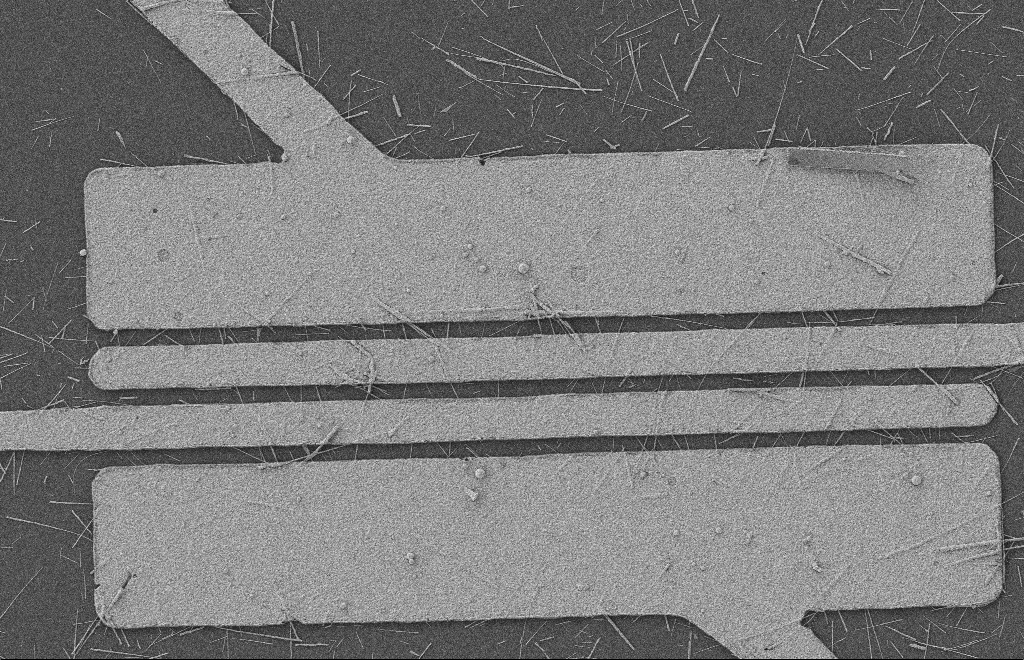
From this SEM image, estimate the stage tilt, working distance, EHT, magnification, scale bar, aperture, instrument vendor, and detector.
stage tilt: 0°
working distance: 9 mm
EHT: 2 kV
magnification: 5.46 K X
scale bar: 2000 nm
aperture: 20 µm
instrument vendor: Zeiss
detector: SE2